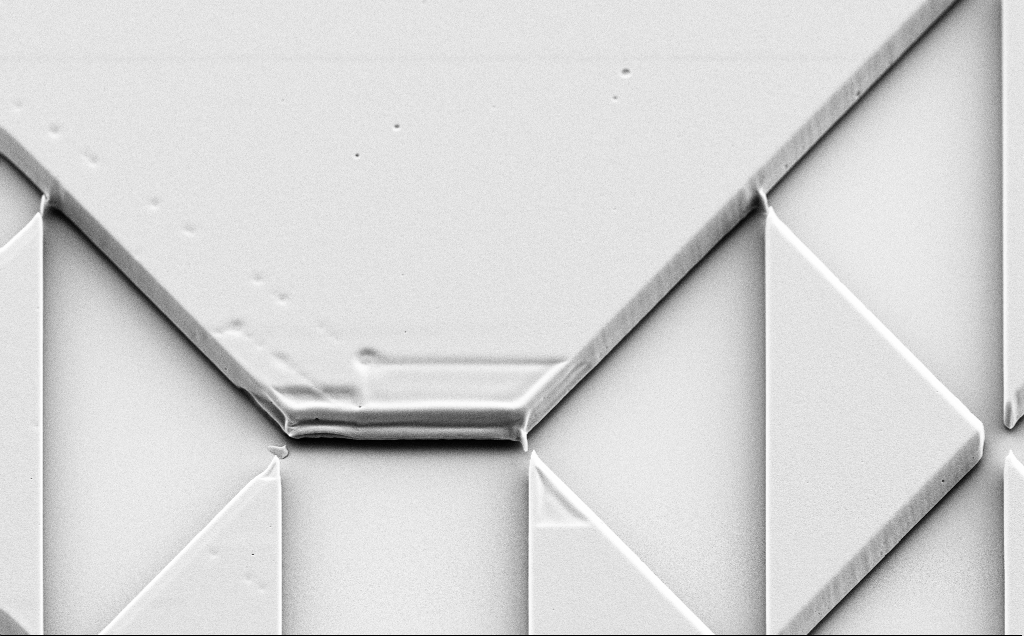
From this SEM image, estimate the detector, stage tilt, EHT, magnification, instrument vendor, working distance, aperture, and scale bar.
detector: SE2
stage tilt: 40°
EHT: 5 kV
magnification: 2.48 K X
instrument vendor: Zeiss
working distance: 9 mm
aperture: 30 µm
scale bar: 10000 nm